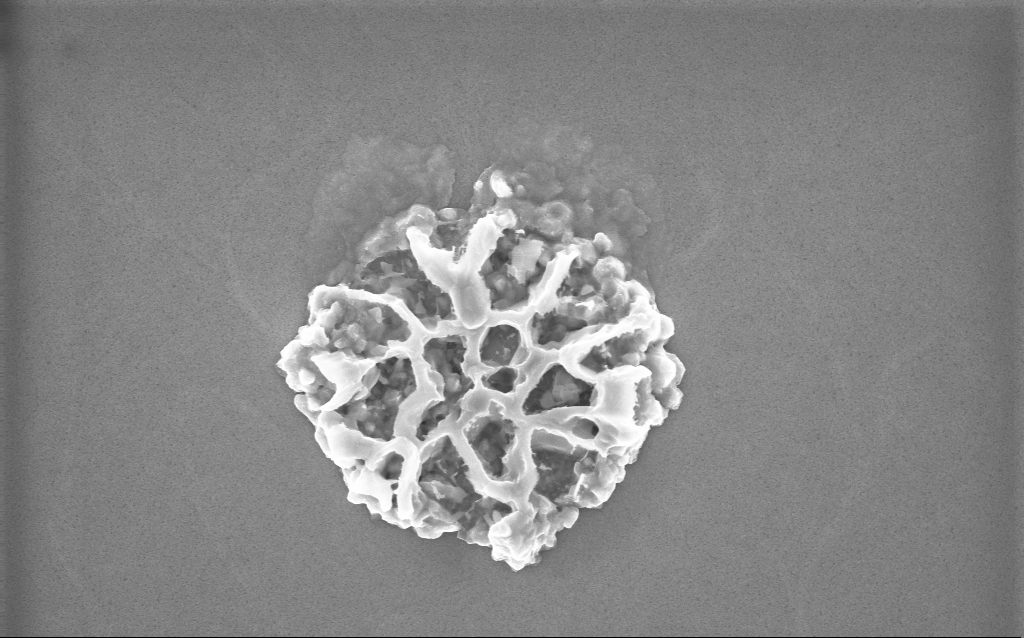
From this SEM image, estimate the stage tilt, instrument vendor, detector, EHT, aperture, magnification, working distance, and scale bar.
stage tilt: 0°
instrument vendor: Zeiss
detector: InLens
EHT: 10 kV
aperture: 30 µm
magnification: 49.15 K X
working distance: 2 mm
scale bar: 1000 nm